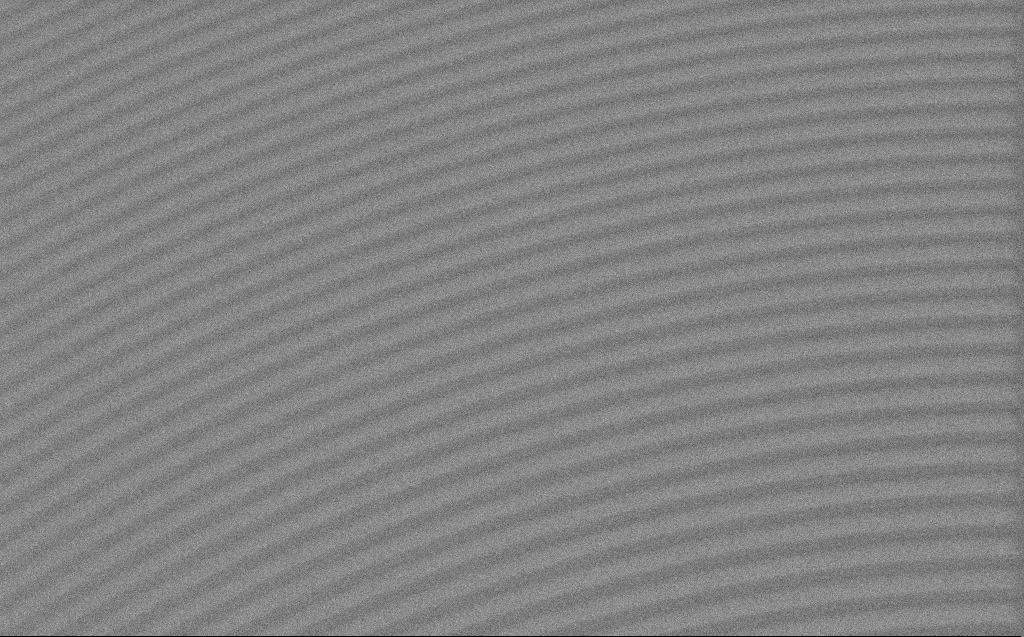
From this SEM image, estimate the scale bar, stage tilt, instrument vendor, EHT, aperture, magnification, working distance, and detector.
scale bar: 2000 nm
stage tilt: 0°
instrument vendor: Zeiss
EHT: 3 kV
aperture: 30 µm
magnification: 12.56 K X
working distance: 4 mm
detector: SE2